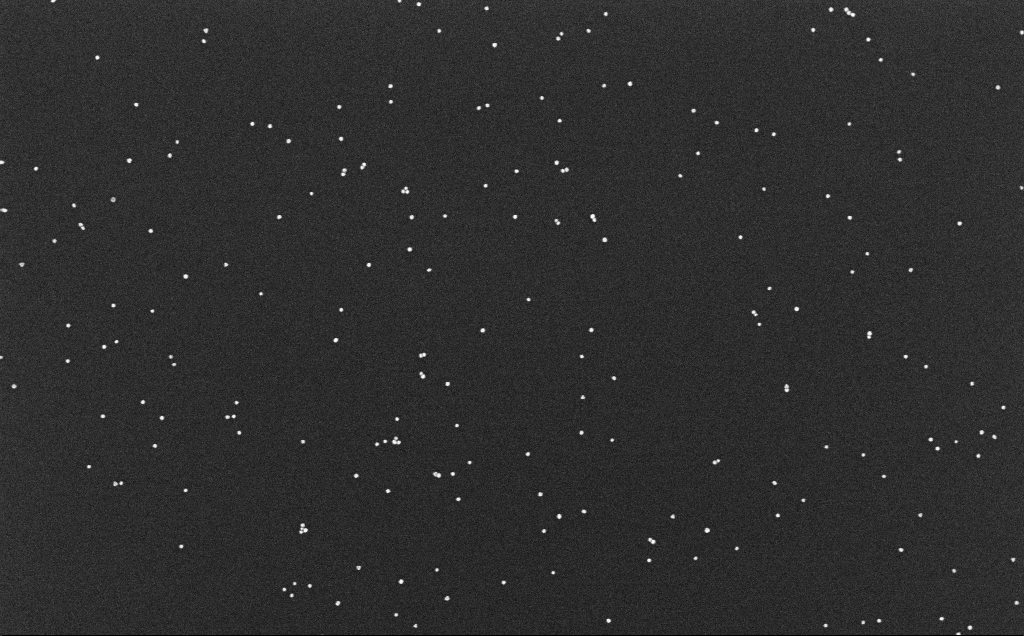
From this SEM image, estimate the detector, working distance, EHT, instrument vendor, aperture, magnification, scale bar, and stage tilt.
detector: InLens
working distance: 6.6 mm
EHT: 10 kV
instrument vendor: Zeiss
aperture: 30 µm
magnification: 100 K X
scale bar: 200 nm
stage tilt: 0°